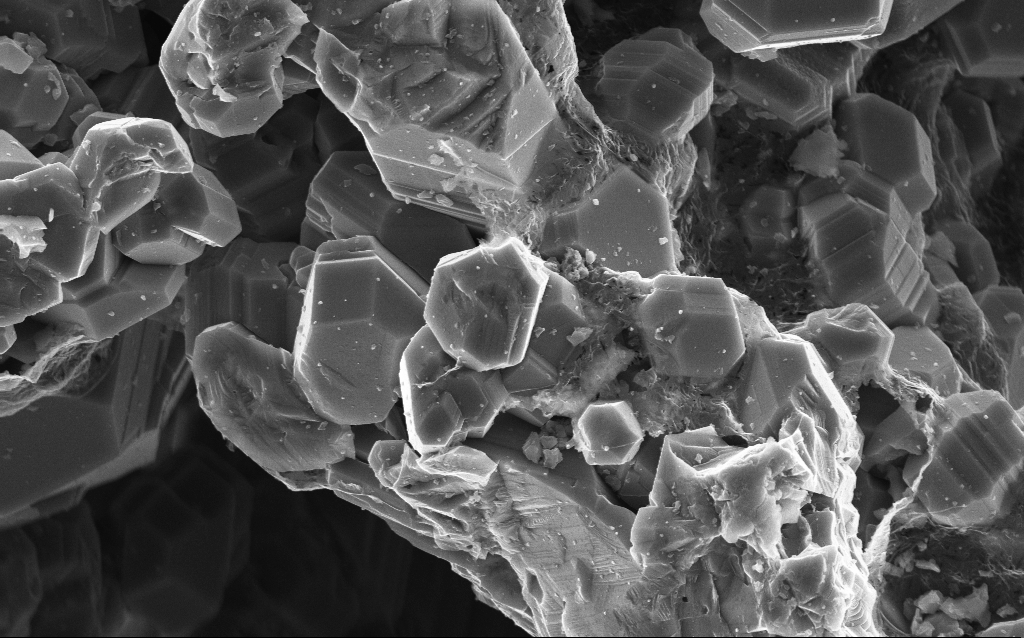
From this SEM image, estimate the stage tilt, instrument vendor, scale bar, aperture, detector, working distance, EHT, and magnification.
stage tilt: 0°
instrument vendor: Zeiss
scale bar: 2000 nm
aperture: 30 µm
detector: InLens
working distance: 3 mm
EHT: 10 kV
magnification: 10 K X